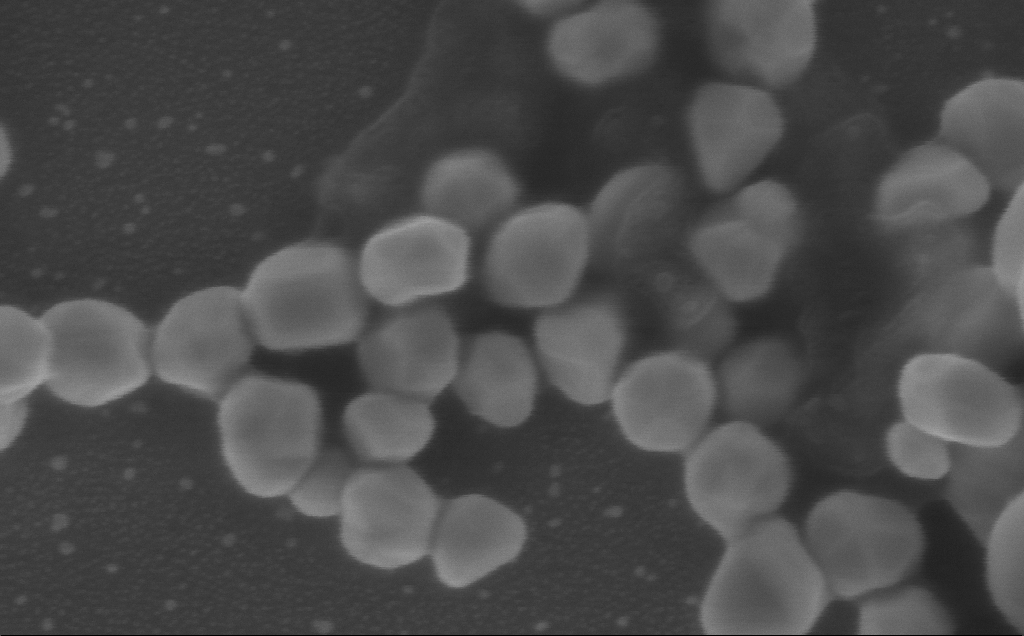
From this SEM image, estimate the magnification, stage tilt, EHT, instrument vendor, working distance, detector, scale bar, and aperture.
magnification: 300 K X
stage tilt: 0°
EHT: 5 kV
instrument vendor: Zeiss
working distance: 3 mm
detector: InLens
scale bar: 100 nm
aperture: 30 µm